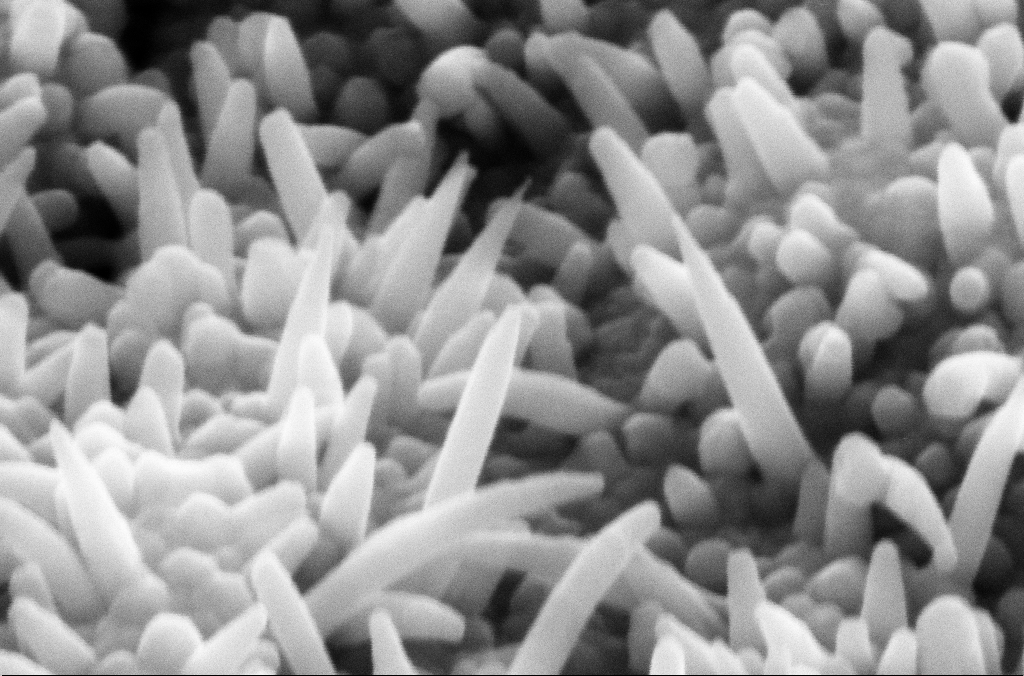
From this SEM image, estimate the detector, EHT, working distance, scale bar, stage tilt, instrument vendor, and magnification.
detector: SE2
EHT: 10 kV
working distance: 6.6 mm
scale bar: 200 nm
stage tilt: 45°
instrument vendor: Zeiss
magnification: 283.27 K X